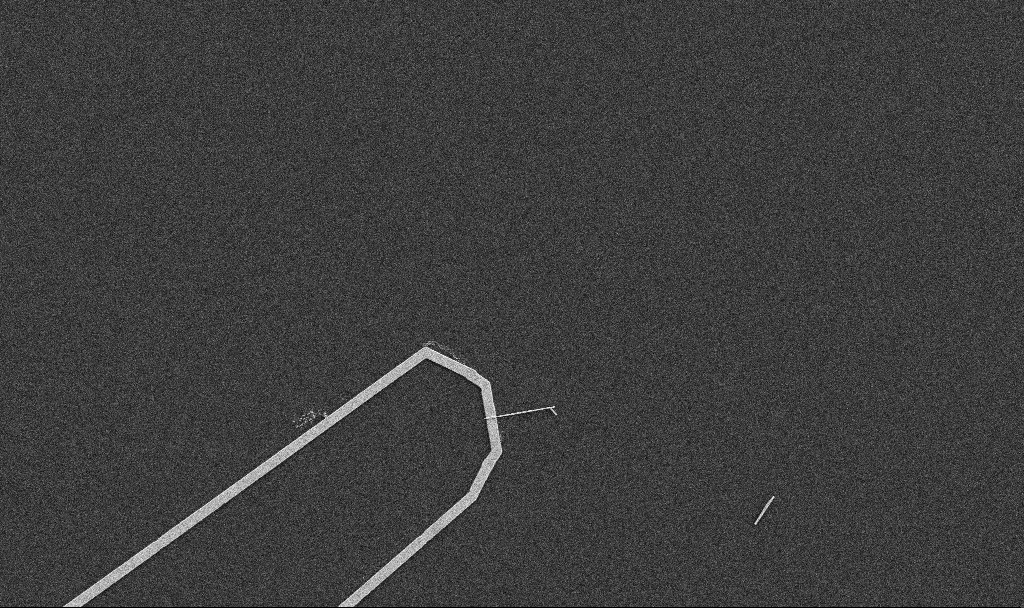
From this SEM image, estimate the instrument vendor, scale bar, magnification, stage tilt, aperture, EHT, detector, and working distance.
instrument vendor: Zeiss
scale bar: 10000 nm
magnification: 5 K X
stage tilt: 0°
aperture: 30 µm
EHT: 5 kV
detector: SE2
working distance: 10.7 mm